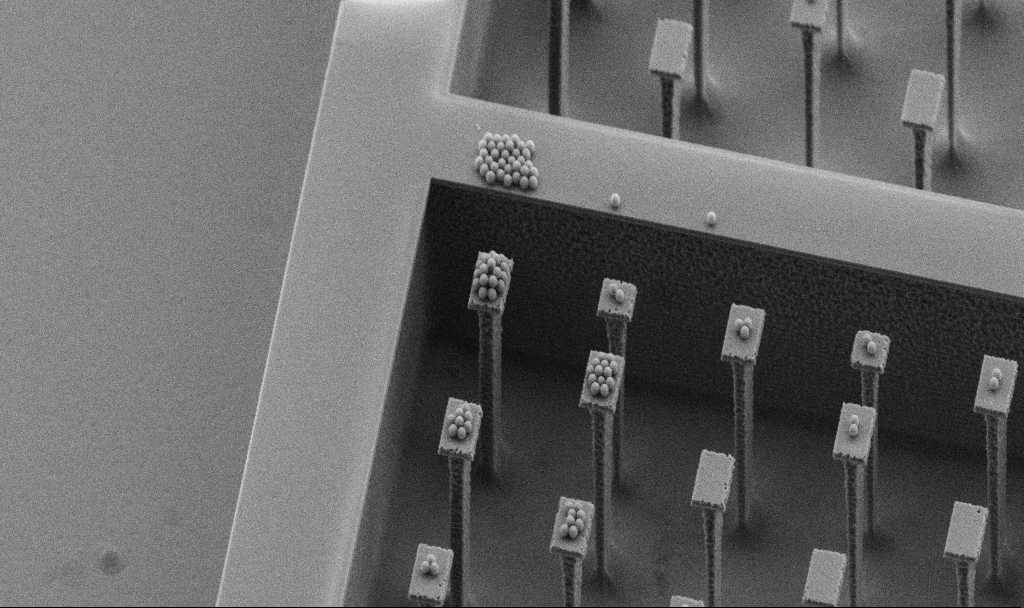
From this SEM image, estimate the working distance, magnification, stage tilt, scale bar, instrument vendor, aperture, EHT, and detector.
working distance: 5.9 mm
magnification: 4.55 K X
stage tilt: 45°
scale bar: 10000 nm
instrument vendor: Zeiss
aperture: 30 µm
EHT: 5 kV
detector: SE2